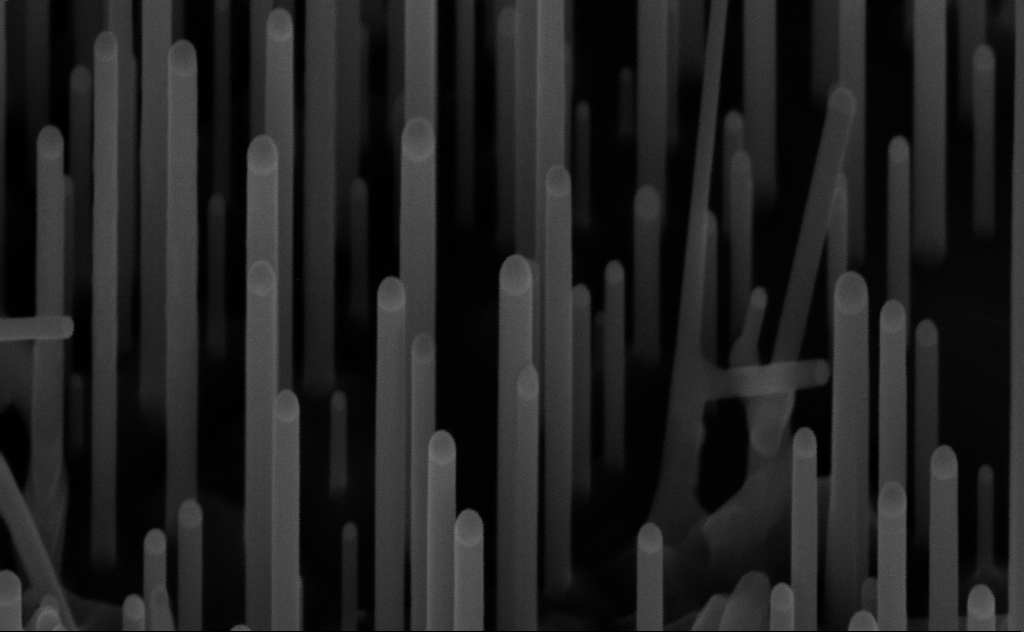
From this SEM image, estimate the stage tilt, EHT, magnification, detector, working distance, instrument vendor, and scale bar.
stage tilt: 45°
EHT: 10 kV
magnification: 173.51 K X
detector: InLens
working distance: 7 mm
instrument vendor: Zeiss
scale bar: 200 nm